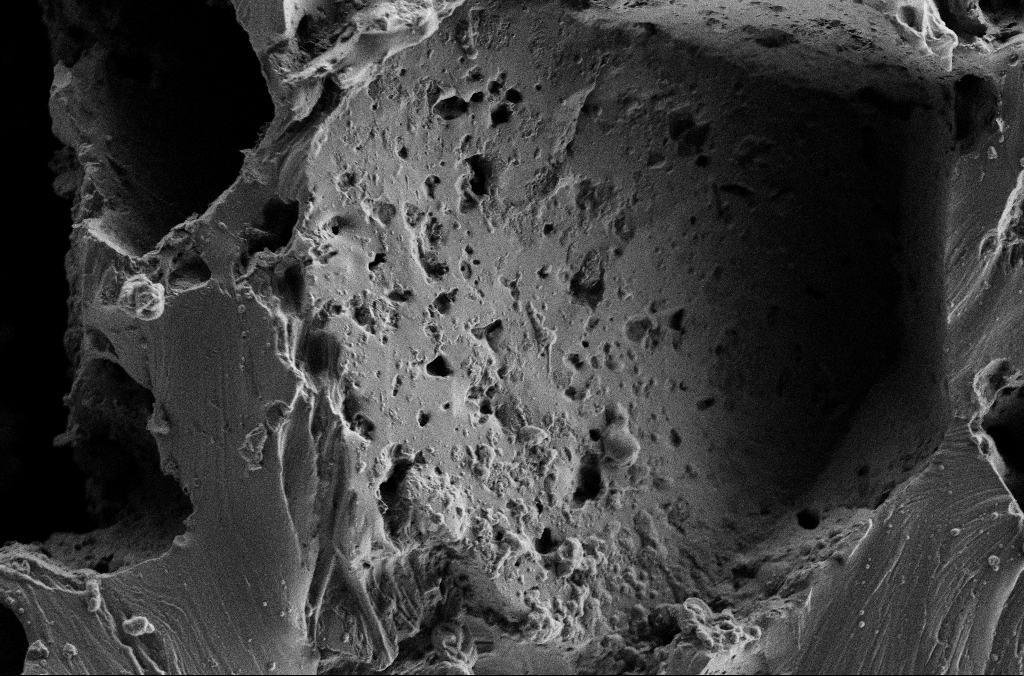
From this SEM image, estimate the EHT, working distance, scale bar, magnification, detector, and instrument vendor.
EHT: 2 kV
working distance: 3 mm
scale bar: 10000 nm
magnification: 2.5 K X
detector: SE2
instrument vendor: Zeiss